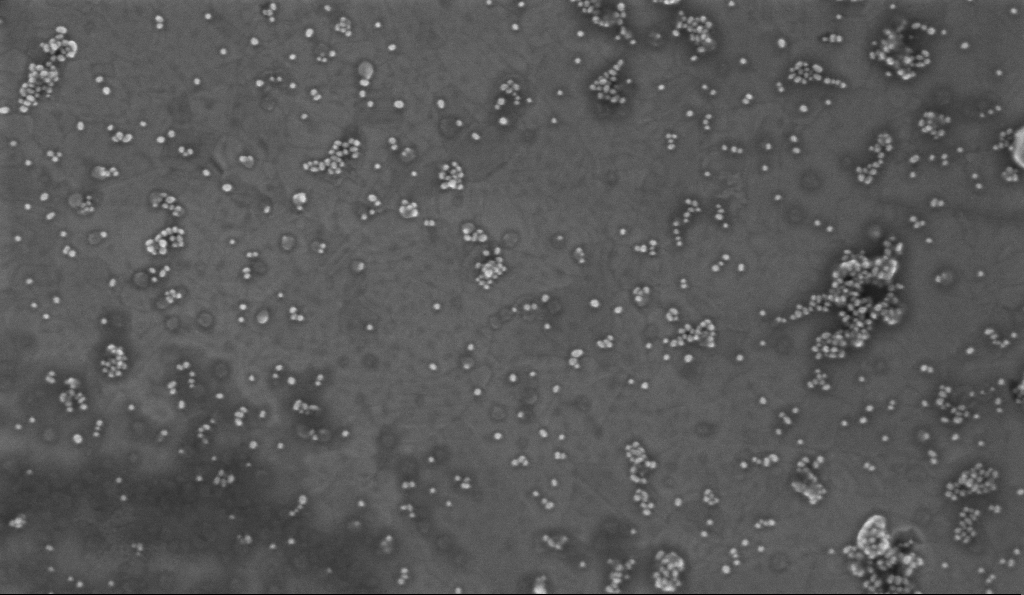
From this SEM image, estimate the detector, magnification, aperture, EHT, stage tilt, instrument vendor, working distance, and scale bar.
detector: InLens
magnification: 100 K X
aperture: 30 µm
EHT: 2 kV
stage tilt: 0°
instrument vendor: Zeiss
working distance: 3.2 mm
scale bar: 200 nm